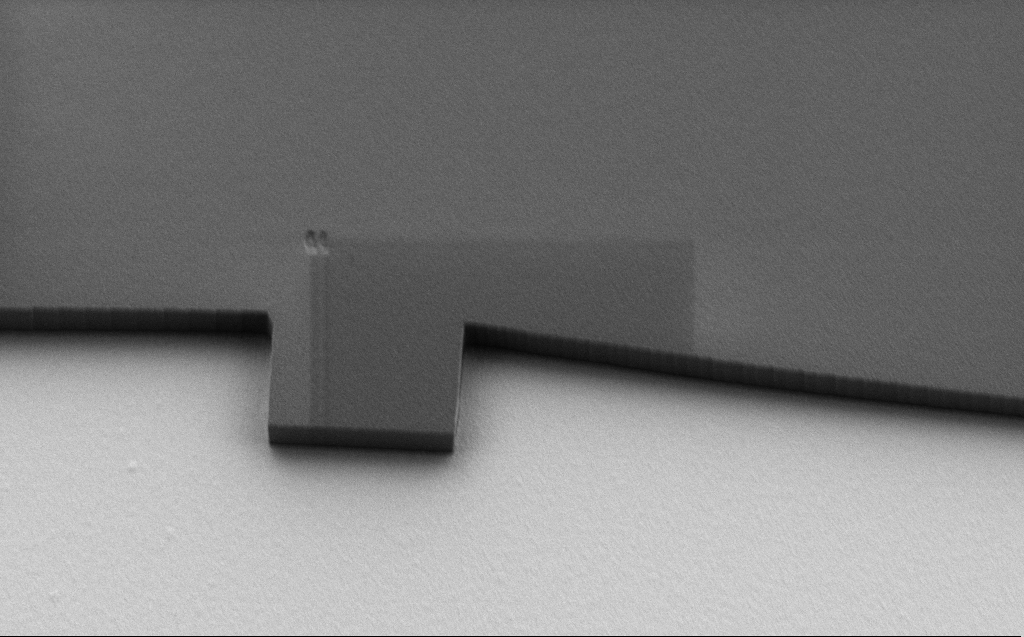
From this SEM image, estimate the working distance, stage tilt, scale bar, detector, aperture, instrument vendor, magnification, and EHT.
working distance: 6 mm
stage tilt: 30°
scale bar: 2000 nm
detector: SE2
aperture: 30 µm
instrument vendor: Zeiss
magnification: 7.28 K X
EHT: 1.1 kV